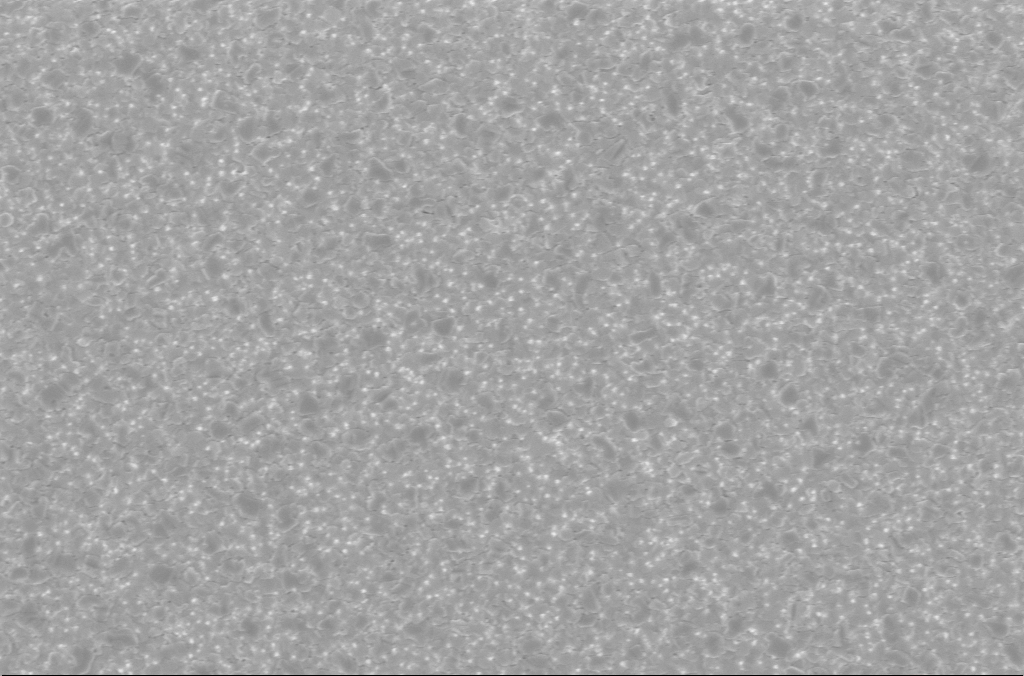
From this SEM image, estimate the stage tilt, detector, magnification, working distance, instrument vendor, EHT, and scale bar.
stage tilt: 0°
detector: InLens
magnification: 15 K X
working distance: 3 mm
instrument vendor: Zeiss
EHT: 5 kV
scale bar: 2000 nm